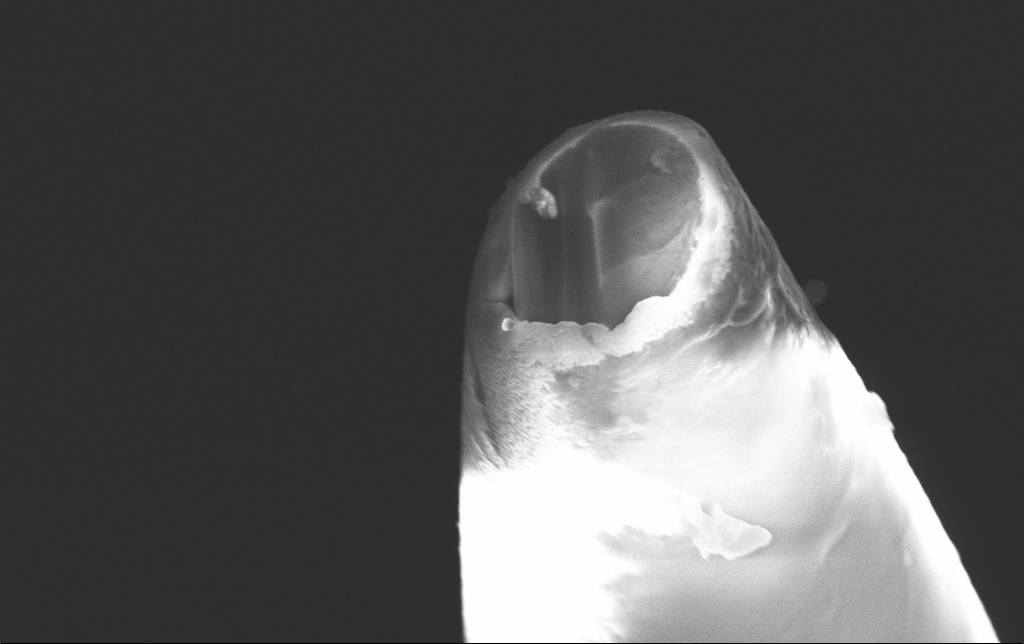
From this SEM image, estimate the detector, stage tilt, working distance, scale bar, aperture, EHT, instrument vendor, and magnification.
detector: InLens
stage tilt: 69.3°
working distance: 9.1 mm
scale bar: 1000 nm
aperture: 30 µm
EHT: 10 kV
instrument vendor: Zeiss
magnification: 41.21 K X